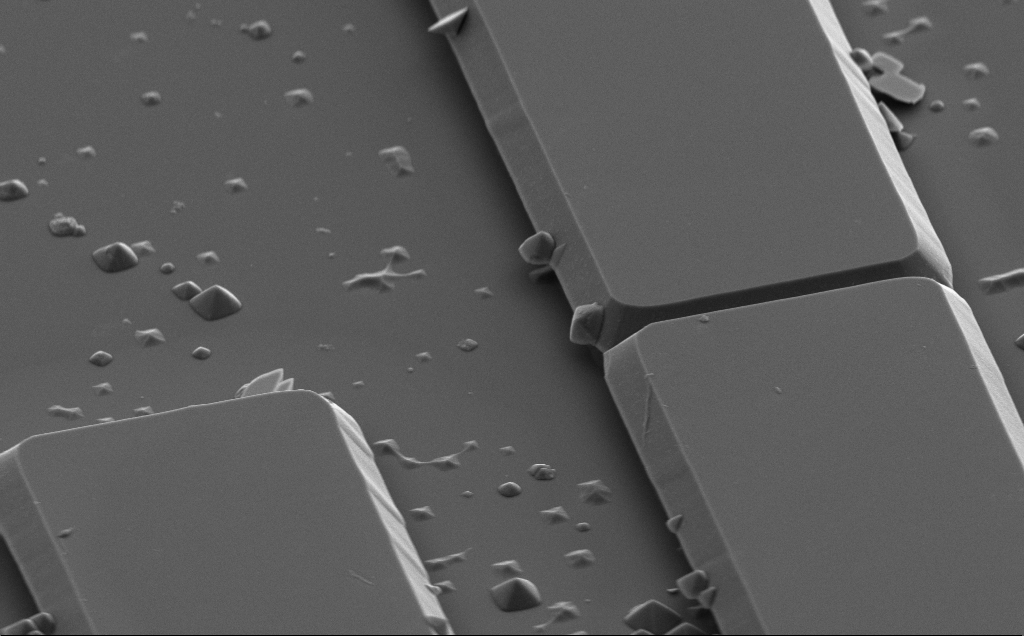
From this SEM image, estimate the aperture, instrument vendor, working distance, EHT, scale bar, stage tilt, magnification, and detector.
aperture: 30 µm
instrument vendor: Zeiss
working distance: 10 mm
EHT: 5 kV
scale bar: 10000 nm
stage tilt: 50°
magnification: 5.87 K X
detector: SE2